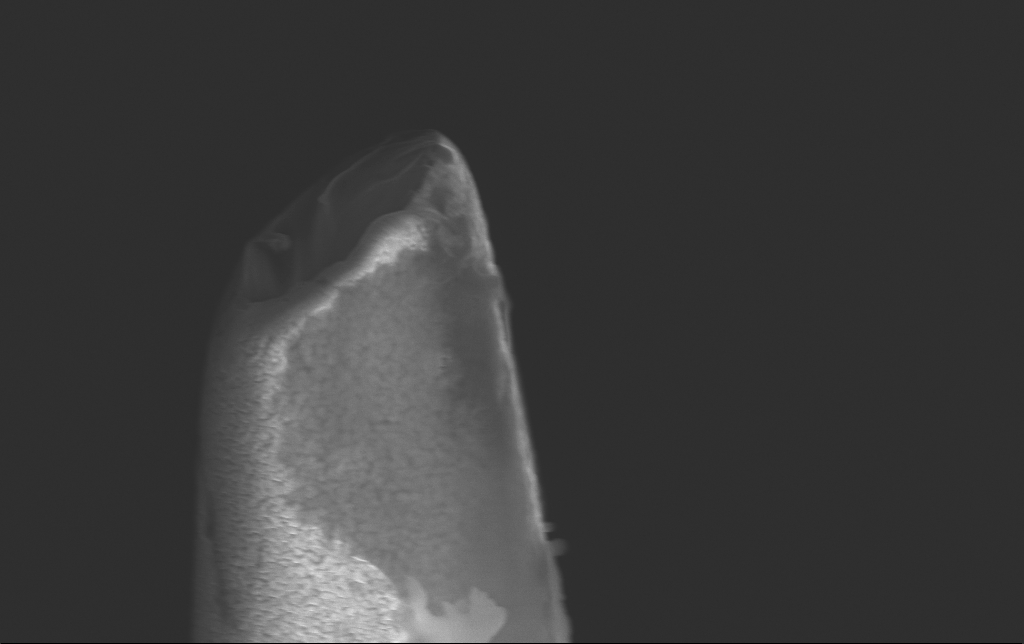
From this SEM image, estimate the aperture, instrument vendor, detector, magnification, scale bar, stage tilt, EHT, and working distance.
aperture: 30 µm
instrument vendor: Zeiss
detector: InLens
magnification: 41 K X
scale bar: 1000 nm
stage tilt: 27.9°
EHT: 10 kV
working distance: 5.3 mm